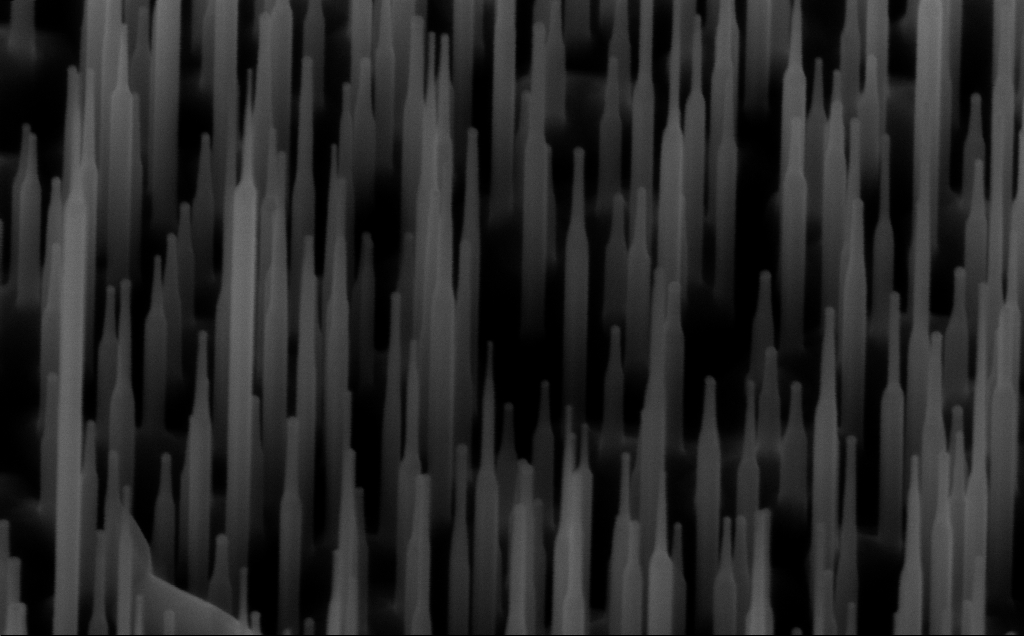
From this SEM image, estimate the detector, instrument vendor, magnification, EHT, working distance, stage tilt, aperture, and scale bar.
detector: InLens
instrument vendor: Zeiss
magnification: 150 K X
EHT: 10 kV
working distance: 6 mm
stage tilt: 45°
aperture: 30 µm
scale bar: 100 nm